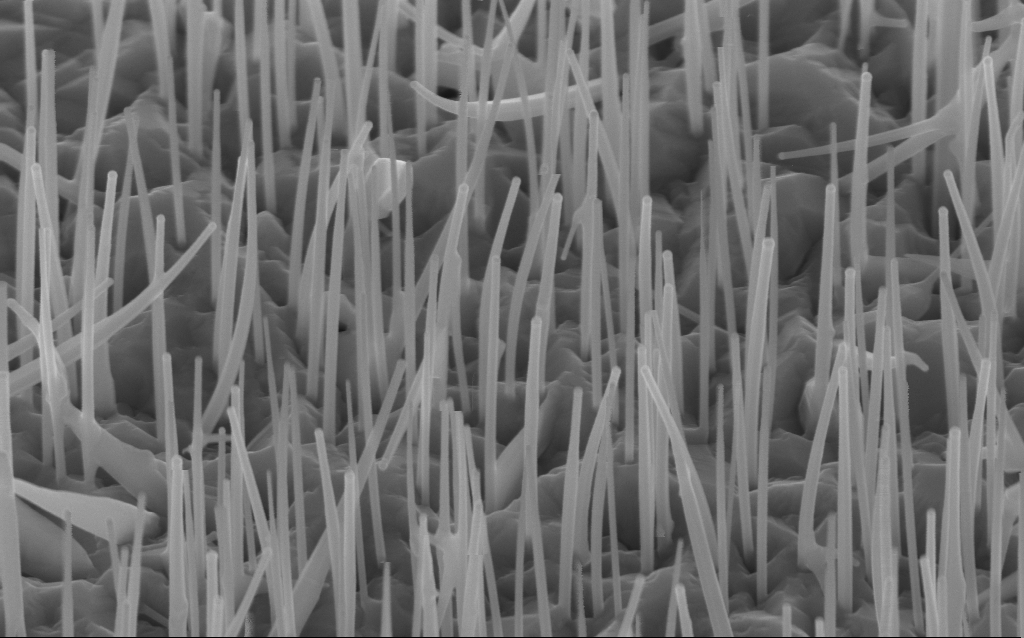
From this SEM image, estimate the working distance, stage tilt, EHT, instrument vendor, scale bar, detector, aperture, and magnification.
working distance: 6 mm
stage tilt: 45°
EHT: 10 kV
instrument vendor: Zeiss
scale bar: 200 nm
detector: InLens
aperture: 30 µm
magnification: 72.6 K X